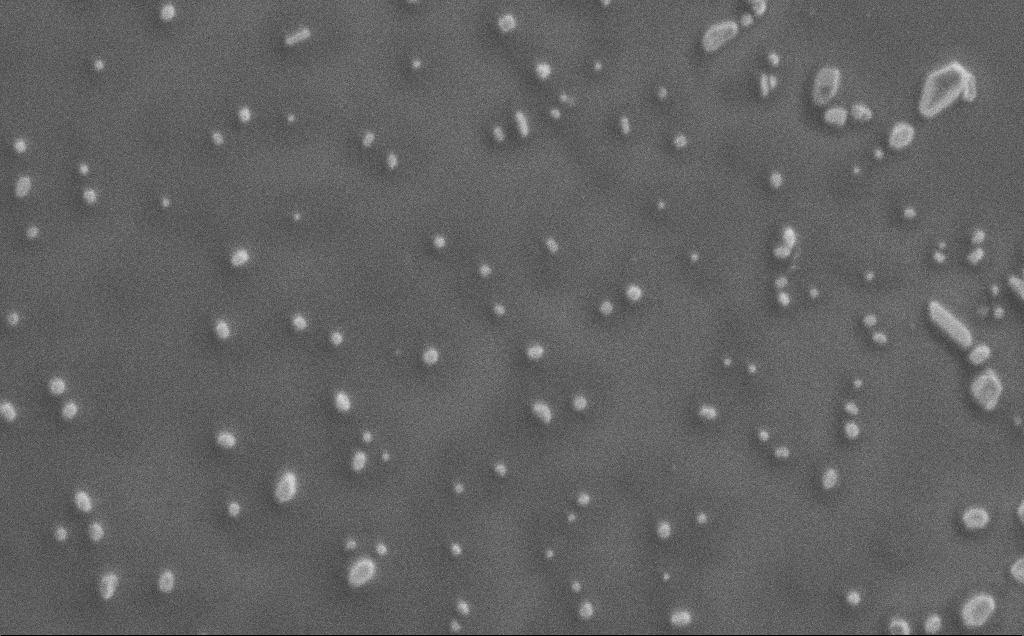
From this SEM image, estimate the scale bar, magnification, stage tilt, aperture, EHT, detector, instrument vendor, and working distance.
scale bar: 2000 nm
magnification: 15.69 K X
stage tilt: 0°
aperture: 30 µm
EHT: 1 kV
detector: SE2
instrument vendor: Zeiss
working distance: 3 mm